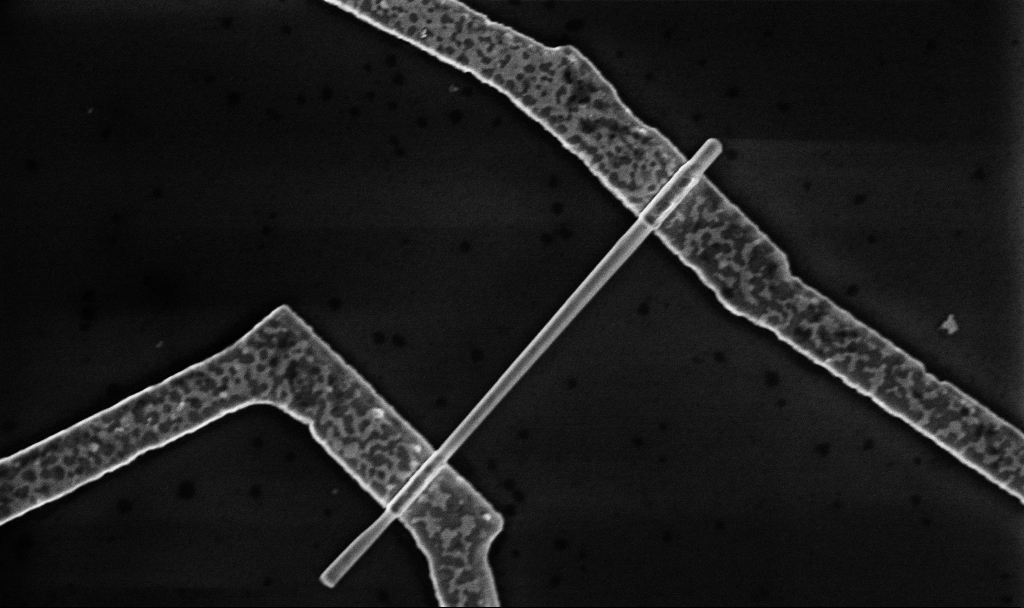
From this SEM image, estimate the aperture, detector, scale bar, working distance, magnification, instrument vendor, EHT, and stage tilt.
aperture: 30 µm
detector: InLens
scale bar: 1000 nm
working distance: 8.7 mm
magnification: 30 K X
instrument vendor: Zeiss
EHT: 5 kV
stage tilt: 0°